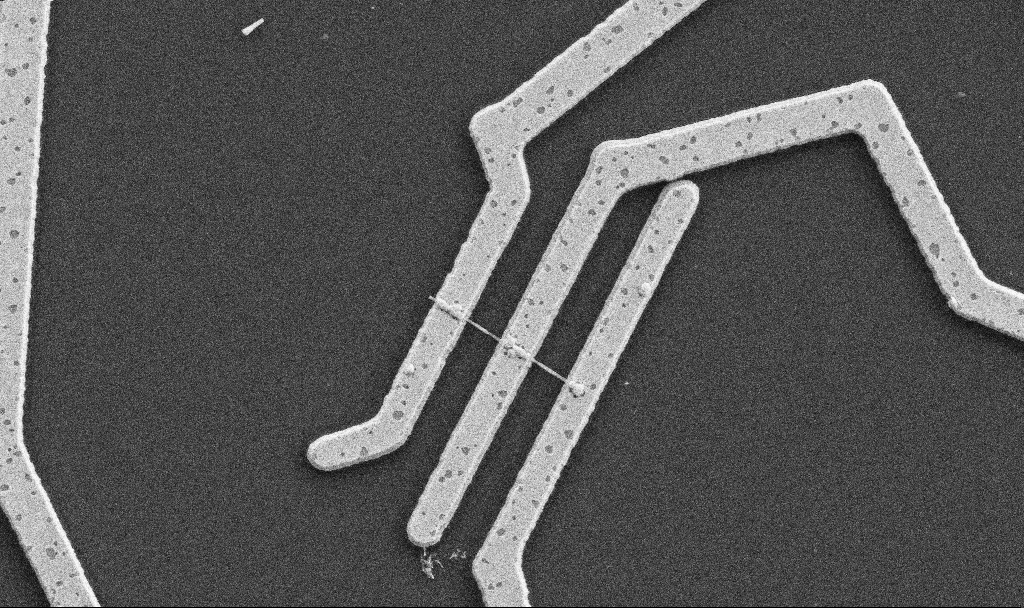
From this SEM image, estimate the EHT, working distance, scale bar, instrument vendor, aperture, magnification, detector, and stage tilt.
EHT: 5 kV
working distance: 10.7 mm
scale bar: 1000 nm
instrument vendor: Zeiss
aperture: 30 µm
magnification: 20 K X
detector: SE2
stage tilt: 0°